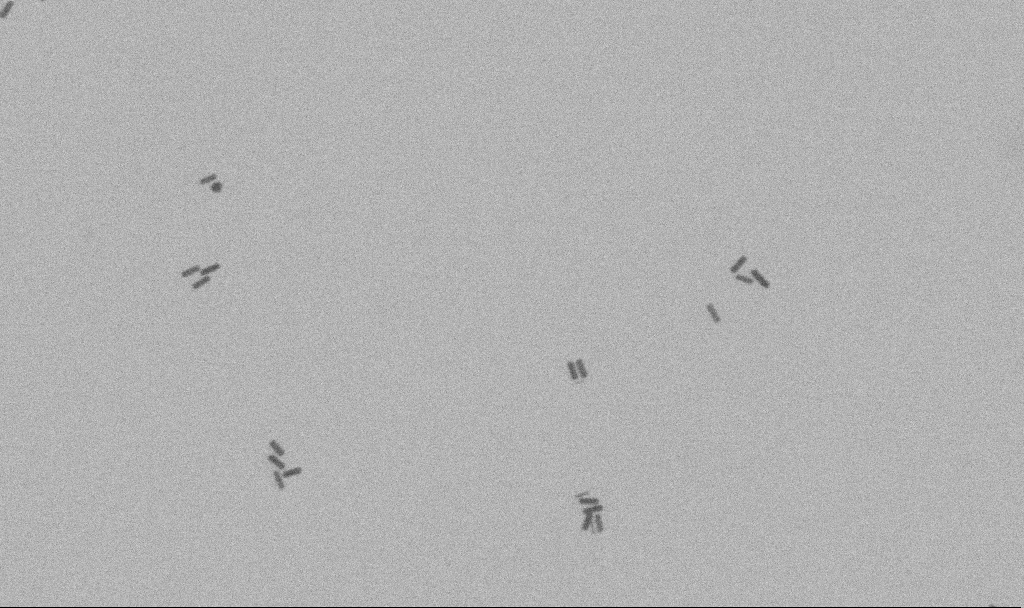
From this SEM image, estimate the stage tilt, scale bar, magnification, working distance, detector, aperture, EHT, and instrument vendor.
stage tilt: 0°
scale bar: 200 nm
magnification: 100 K X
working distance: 11.3 mm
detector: SE2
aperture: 30 µm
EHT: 10 kV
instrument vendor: Zeiss